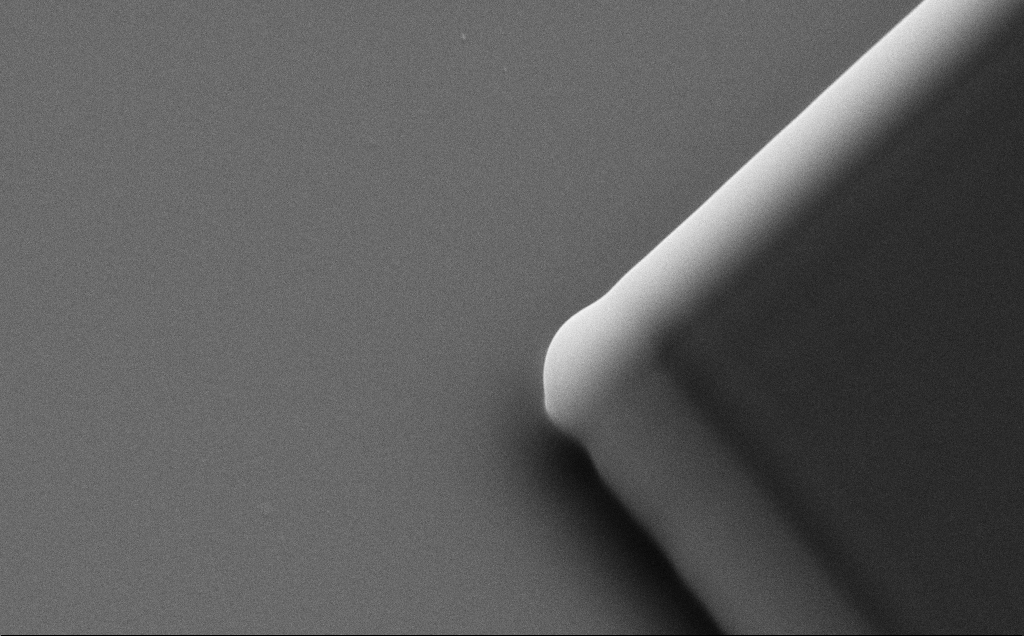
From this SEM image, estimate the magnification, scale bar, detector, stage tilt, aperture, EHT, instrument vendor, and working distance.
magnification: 23.33 K X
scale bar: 2000 nm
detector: SE2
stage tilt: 35°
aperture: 30 µm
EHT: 10 kV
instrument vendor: Zeiss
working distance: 8 mm